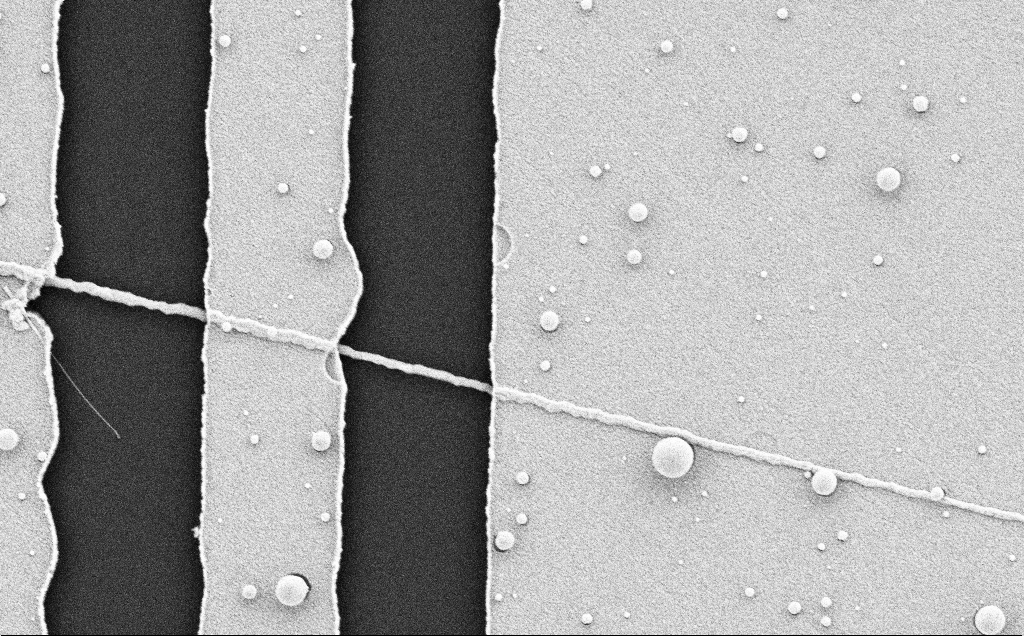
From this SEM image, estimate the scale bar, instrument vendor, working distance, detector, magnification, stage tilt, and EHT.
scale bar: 2000 nm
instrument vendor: Zeiss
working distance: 8 mm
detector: SE2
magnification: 26.66 K X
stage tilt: -0.7°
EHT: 5 kV